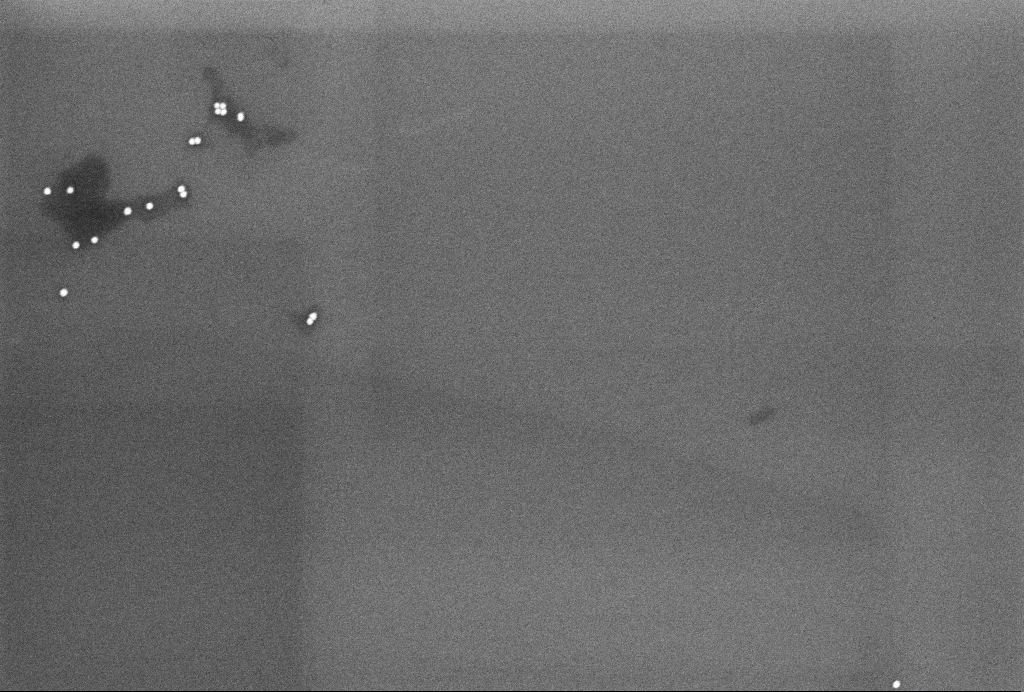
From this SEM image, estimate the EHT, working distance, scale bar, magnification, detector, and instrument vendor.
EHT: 4 kV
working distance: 3.2 mm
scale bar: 200 nm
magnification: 95 K X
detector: InLens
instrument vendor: Zeiss